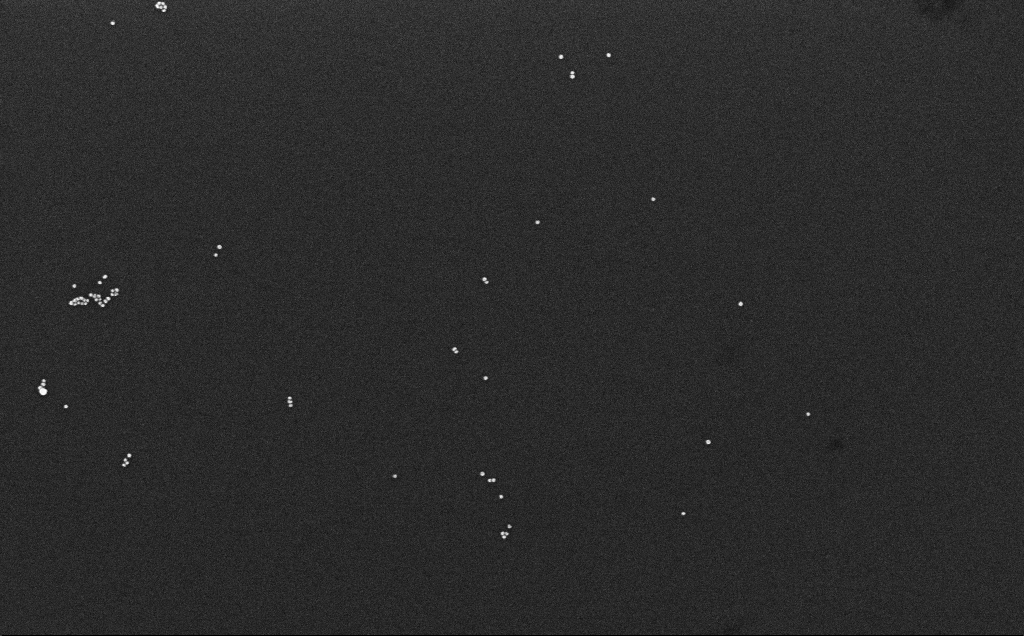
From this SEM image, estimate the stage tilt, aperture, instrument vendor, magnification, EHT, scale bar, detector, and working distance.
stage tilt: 0°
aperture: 30 µm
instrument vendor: Zeiss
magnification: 100 K X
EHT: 10 kV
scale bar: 200 nm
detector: InLens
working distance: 3.3 mm